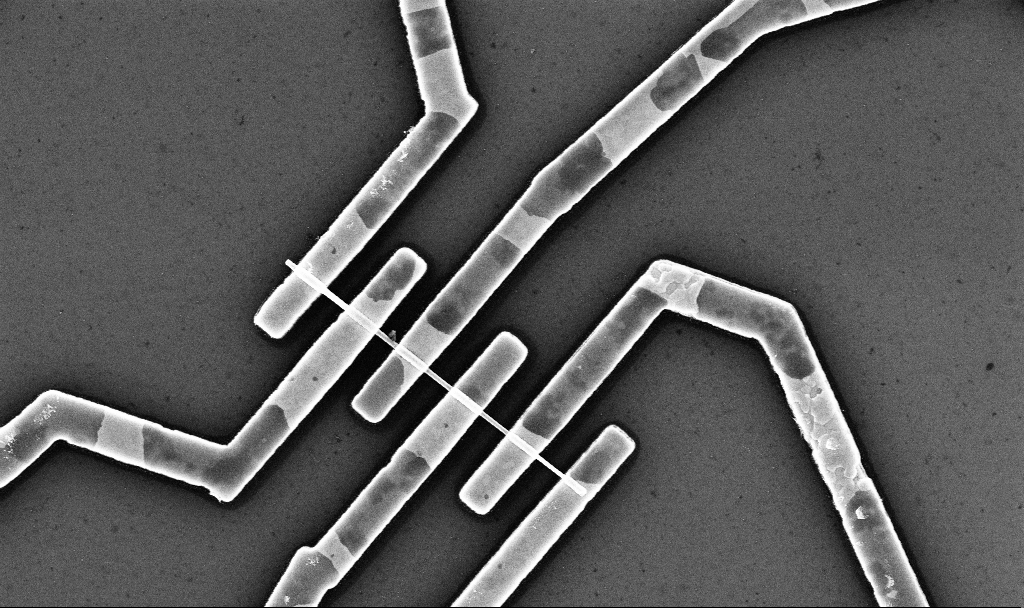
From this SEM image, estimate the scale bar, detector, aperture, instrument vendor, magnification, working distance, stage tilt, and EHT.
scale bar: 2000 nm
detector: InLens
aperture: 30 µm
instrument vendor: Zeiss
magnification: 22.21 K X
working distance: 6.8 mm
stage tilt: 0°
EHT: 10 kV